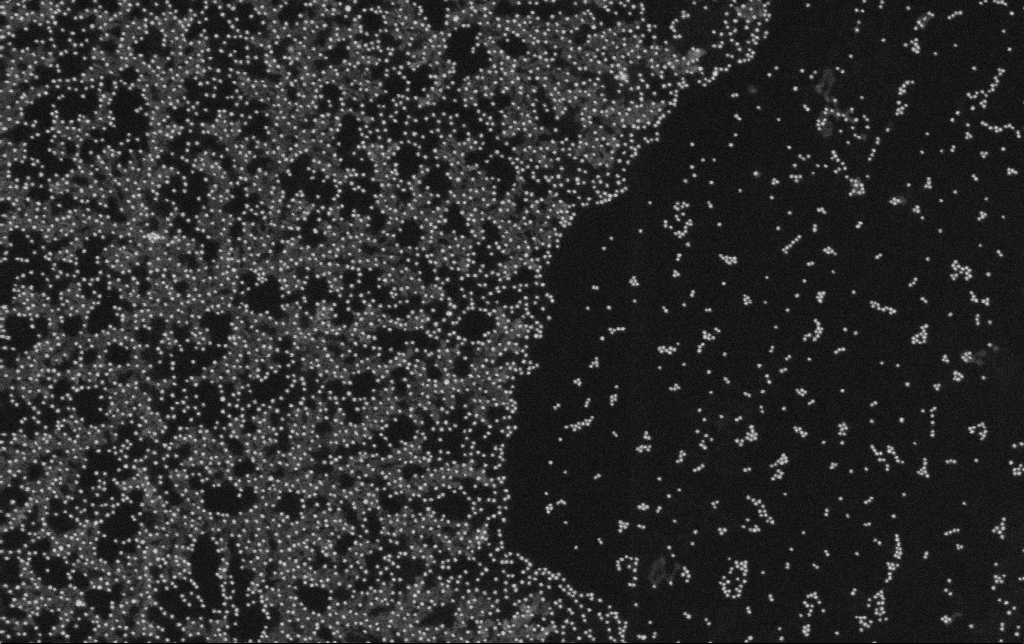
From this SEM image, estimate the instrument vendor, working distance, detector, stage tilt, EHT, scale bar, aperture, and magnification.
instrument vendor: Zeiss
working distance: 11.3 mm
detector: SE2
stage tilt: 0°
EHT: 10 kV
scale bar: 200 nm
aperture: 30 µm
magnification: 100 K X